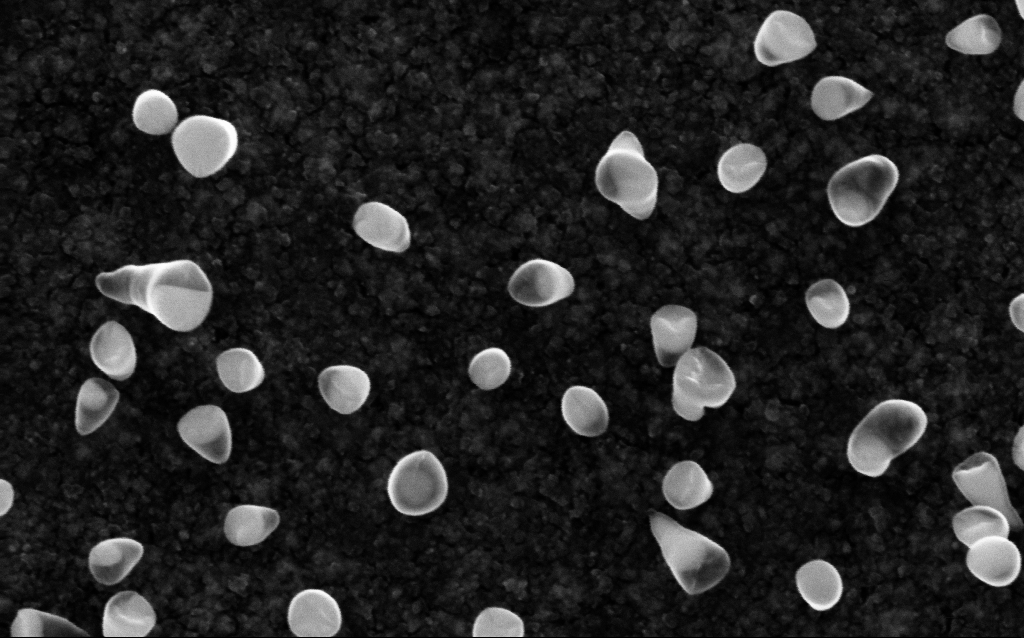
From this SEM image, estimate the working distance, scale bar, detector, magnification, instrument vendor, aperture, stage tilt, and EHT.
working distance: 1.9 mm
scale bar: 100 nm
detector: InLens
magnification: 200 K X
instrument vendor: Zeiss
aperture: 30 µm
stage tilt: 0°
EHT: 5 kV